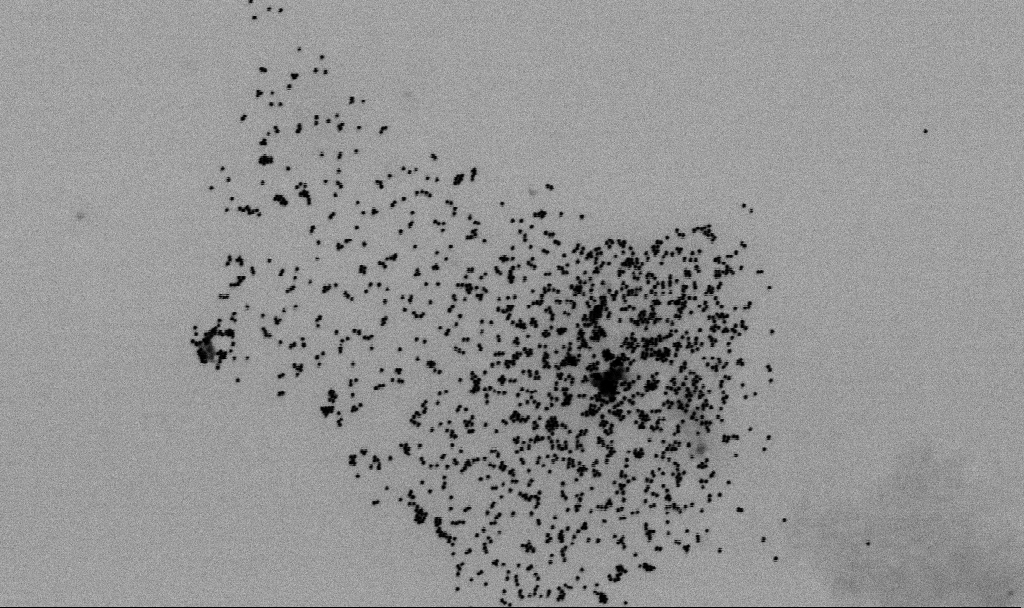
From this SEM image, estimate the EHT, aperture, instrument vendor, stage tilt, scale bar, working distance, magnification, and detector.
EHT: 2 kV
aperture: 30 µm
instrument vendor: Zeiss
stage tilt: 0°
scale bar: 1000 nm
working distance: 6.5 mm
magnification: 62.55 K X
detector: SE2